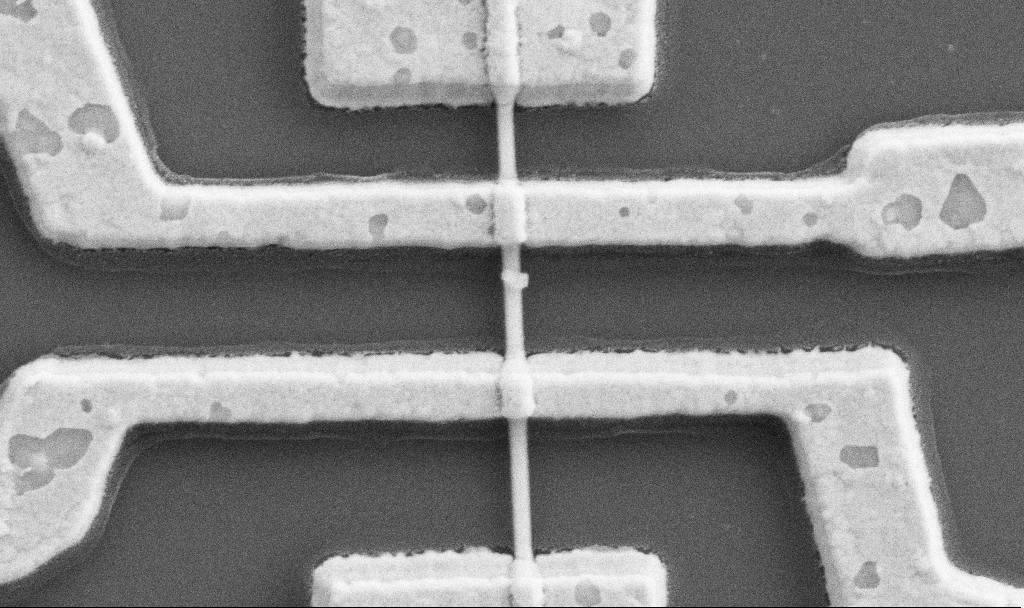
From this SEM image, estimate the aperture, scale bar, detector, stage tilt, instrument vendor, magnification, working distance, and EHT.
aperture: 30 µm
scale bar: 1000 nm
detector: SE2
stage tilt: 0°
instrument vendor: Zeiss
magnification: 60 K X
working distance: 9.7 mm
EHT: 5 kV